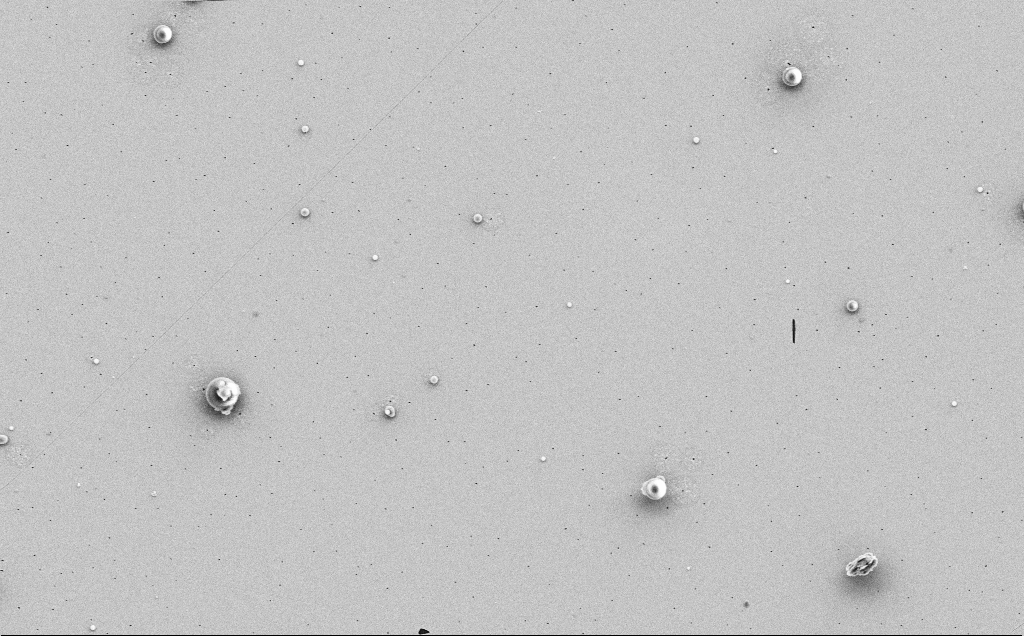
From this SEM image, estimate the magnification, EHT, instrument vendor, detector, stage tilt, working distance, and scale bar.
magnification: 4.2 K X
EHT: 5 kV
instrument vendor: Zeiss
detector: SE2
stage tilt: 0°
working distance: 12 mm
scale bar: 10000 nm